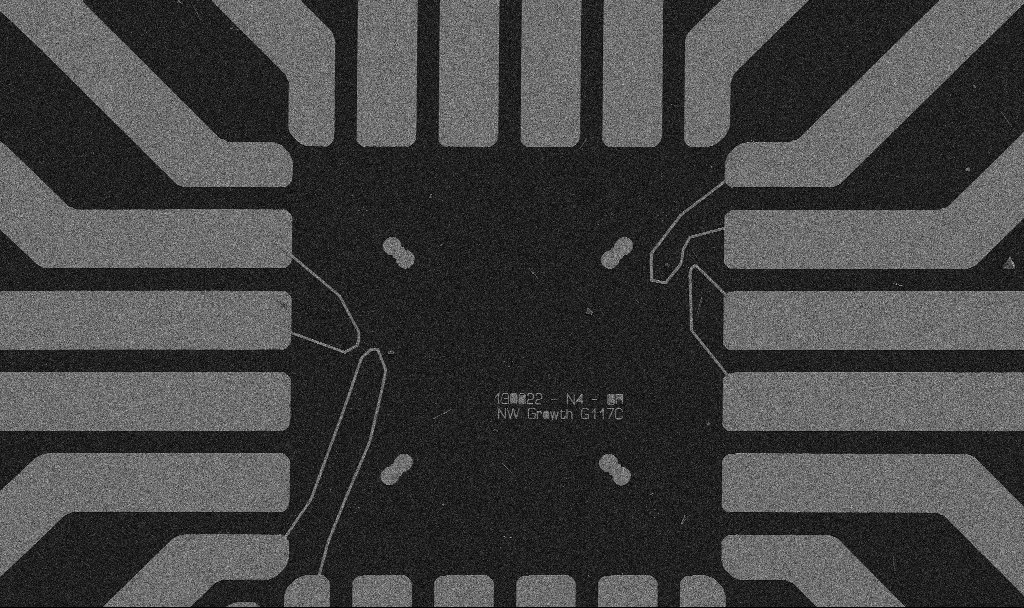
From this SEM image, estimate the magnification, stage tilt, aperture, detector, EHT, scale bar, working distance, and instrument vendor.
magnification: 1 K X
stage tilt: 0°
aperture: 30 µm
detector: SE2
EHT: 5 kV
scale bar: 20000 nm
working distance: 10.7 mm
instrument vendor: Zeiss